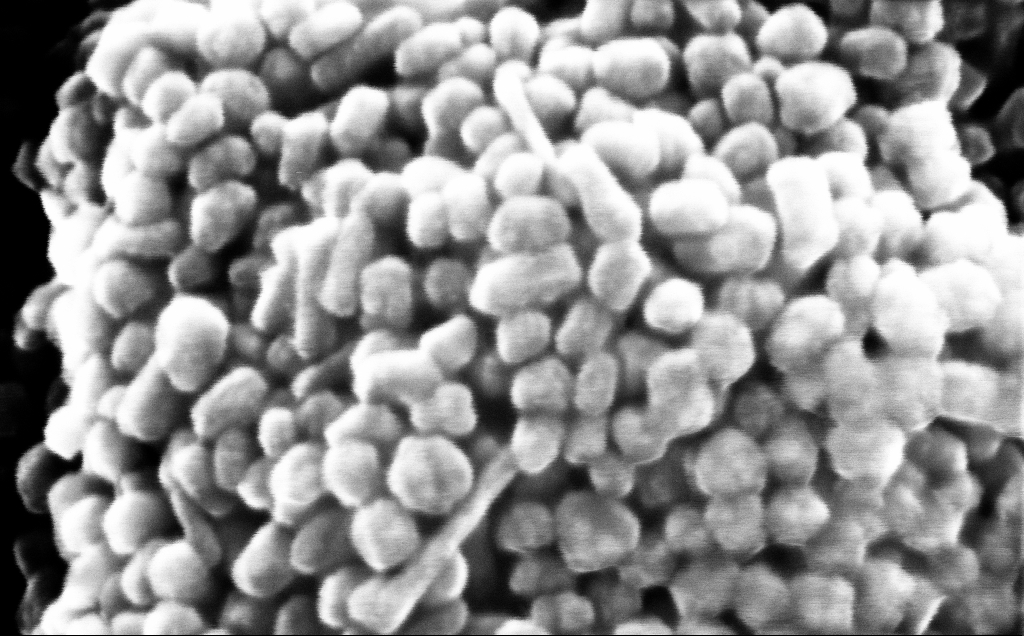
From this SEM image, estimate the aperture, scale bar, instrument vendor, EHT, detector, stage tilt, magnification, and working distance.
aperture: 30 µm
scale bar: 100 nm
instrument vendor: Zeiss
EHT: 20 kV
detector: InLens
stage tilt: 0°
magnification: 669.01 K X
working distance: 3 mm